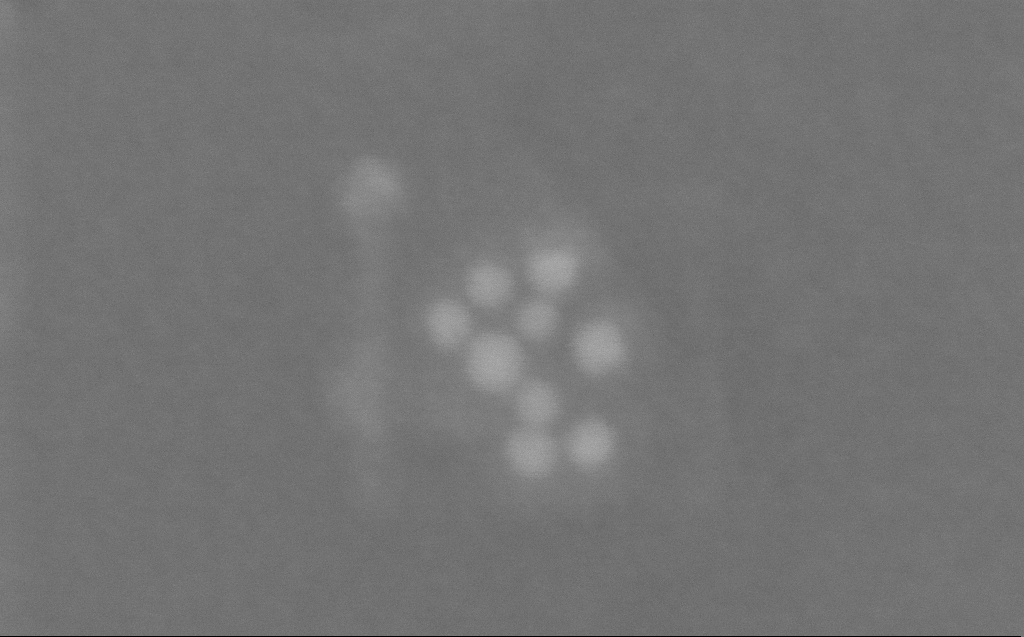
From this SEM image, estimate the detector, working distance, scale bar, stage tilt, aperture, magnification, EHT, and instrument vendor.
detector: InLens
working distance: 3 mm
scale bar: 20 nm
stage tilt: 0°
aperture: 30 µm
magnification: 1159.8 K X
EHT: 10 kV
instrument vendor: Zeiss